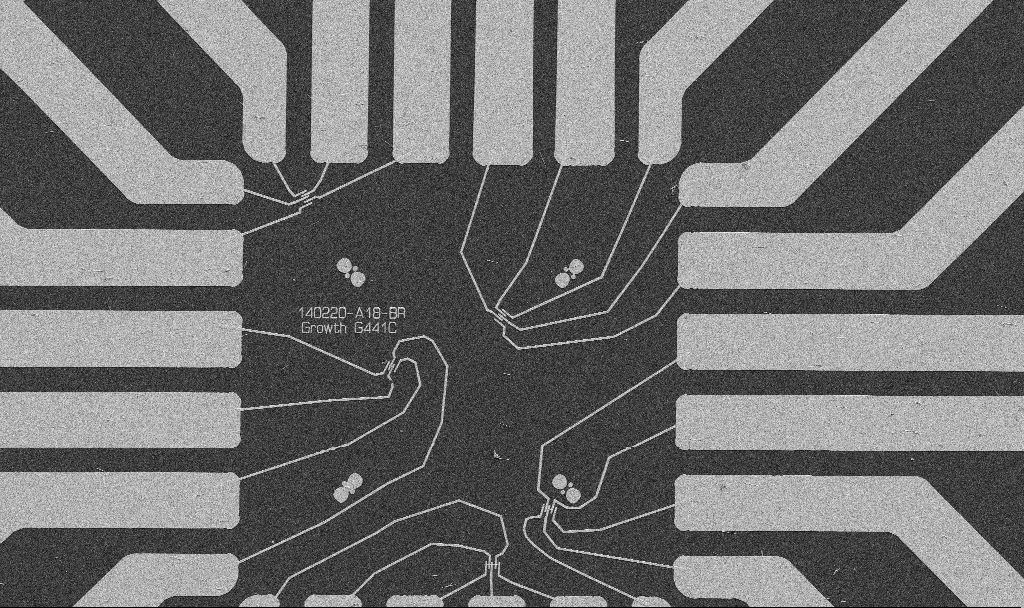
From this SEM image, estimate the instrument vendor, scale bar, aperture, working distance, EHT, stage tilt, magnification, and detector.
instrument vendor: Zeiss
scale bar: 20000 nm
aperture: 30 µm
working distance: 10.7 mm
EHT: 5 kV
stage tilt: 0°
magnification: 1 K X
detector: SE2